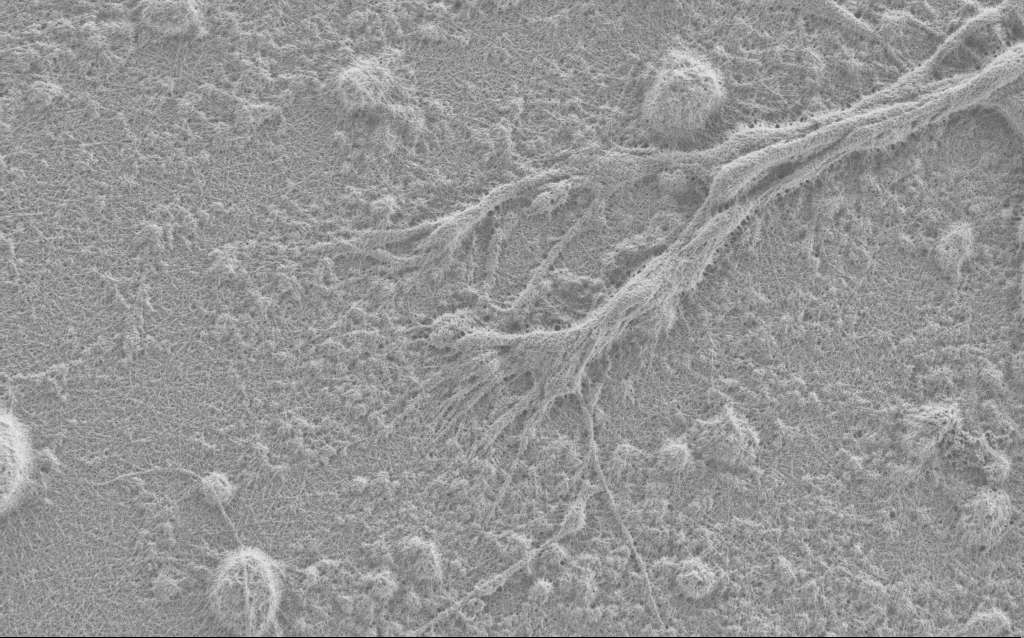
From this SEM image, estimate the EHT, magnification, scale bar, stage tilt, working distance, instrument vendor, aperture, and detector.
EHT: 0.9 kV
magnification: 10 K X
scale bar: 2000 nm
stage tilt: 0°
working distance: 4 mm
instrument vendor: Zeiss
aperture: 30 µm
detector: SE2